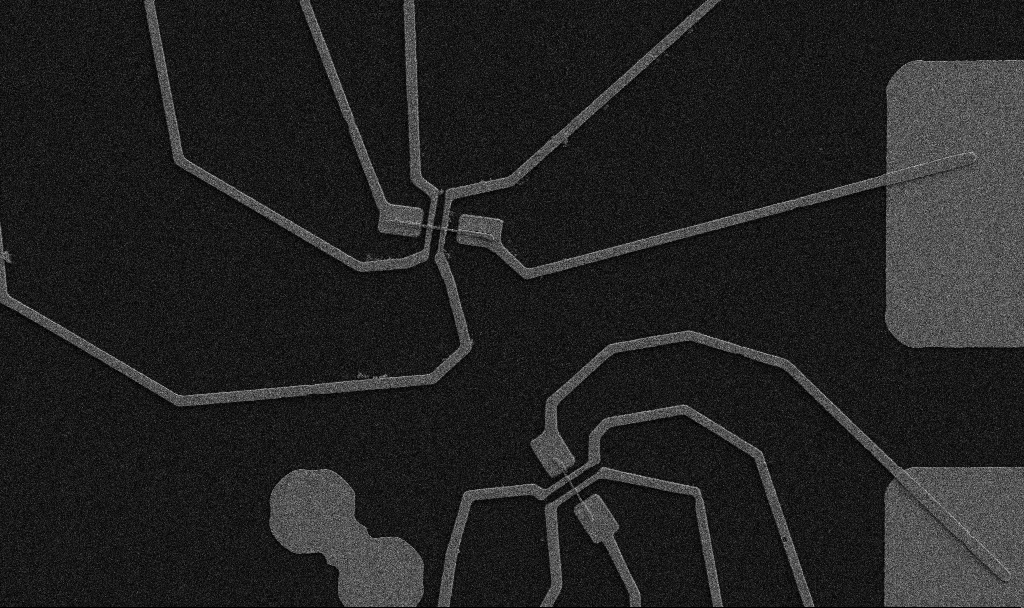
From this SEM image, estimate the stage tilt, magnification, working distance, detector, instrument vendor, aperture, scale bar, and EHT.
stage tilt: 0°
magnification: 5 K X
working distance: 10.7 mm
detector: SE2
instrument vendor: Zeiss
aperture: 30 µm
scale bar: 10000 nm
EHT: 5 kV